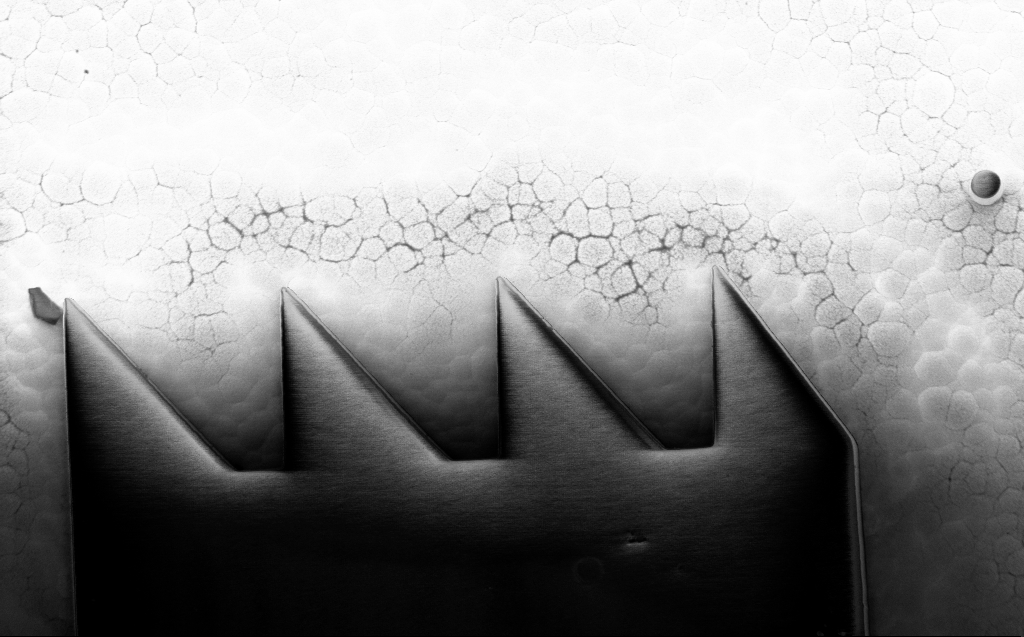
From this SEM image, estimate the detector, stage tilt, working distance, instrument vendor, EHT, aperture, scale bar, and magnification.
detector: InLens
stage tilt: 0°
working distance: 6 mm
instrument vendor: Zeiss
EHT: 1 kV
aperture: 30 µm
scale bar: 10000 nm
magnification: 6.88 K X